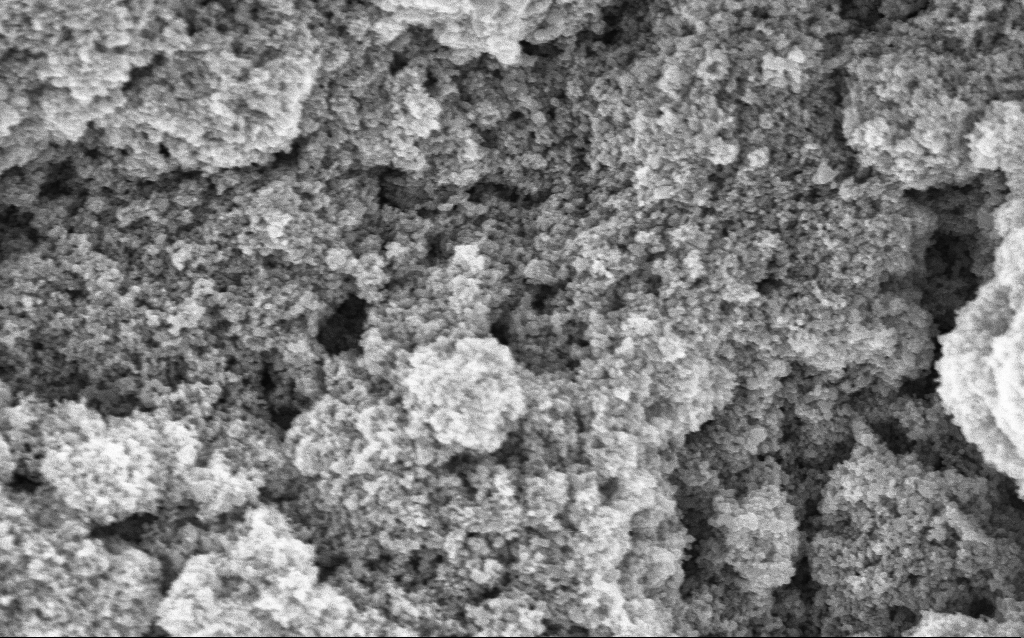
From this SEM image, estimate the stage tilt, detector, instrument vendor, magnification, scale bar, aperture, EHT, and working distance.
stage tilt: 0°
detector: InLens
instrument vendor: Zeiss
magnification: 68.7 K X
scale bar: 1000 nm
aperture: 30 µm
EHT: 5 kV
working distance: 4.6 mm